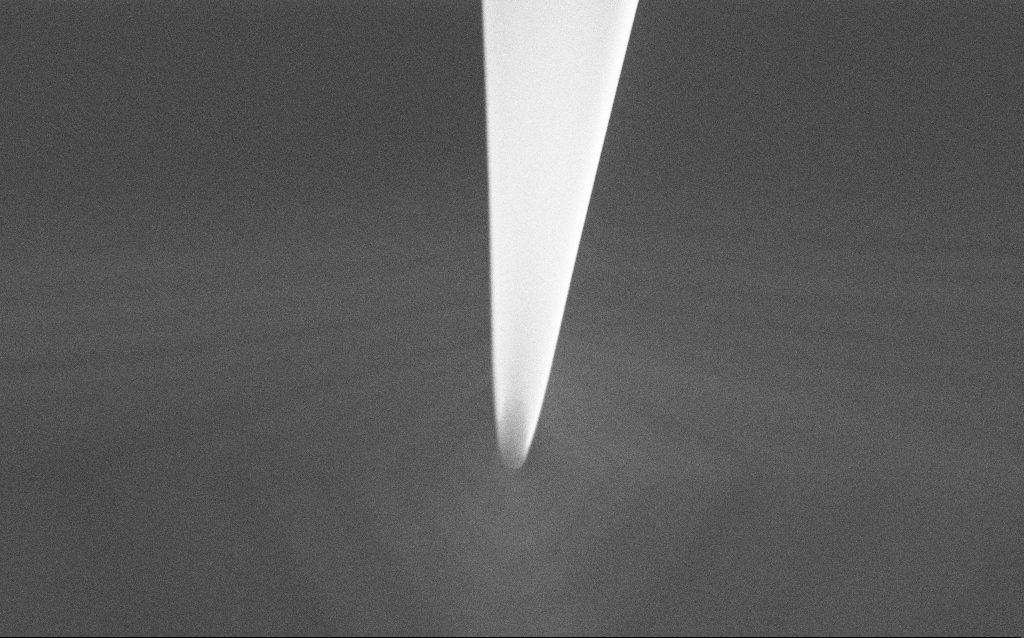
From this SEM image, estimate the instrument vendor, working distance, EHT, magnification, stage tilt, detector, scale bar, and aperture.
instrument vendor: Zeiss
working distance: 5 mm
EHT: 5 kV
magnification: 41.46 K X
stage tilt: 45°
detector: SE2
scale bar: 1000 nm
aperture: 30 µm